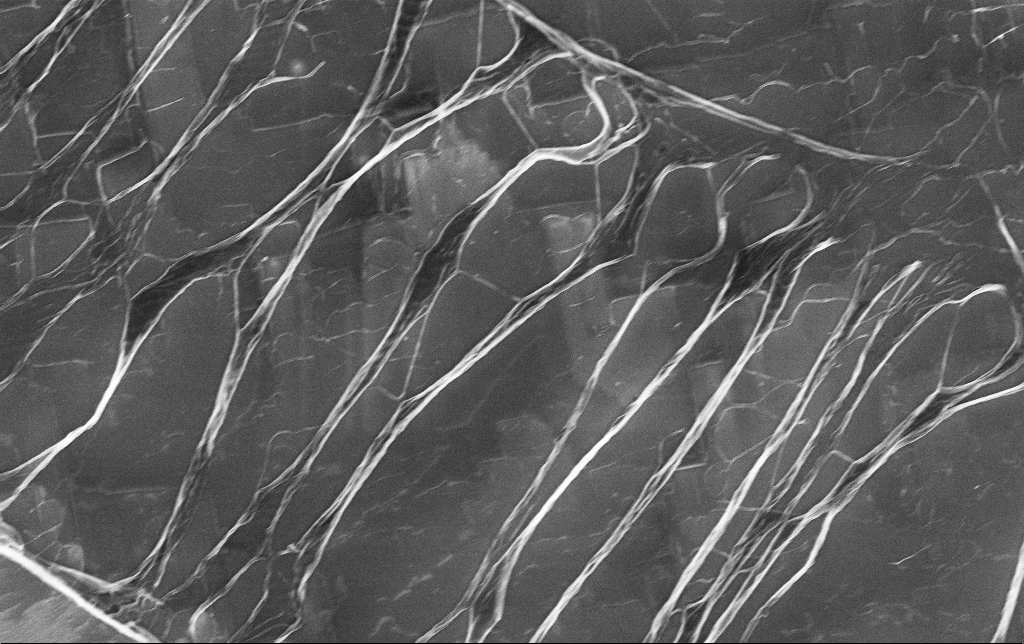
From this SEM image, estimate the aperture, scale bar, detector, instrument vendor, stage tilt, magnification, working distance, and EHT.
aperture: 30 µm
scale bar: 10000 nm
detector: InLens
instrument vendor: Zeiss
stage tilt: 0°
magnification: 6.99 K X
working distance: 3.8 mm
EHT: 5 kV